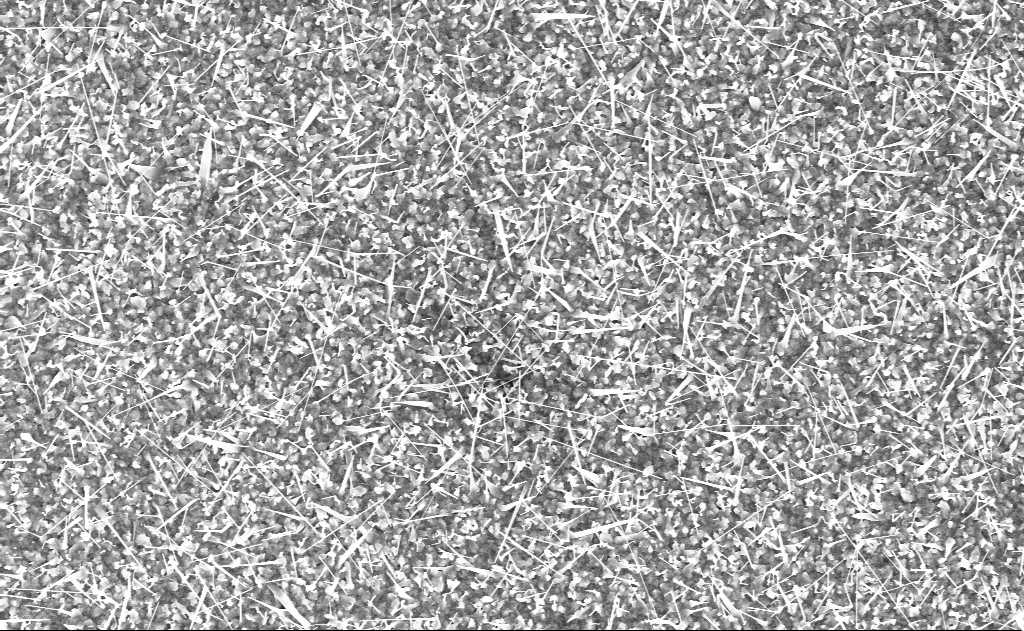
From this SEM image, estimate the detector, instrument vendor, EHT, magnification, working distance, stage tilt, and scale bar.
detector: InLens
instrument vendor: Zeiss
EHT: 10 kV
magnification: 10 K X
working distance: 12 mm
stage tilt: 0°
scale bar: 2000 nm